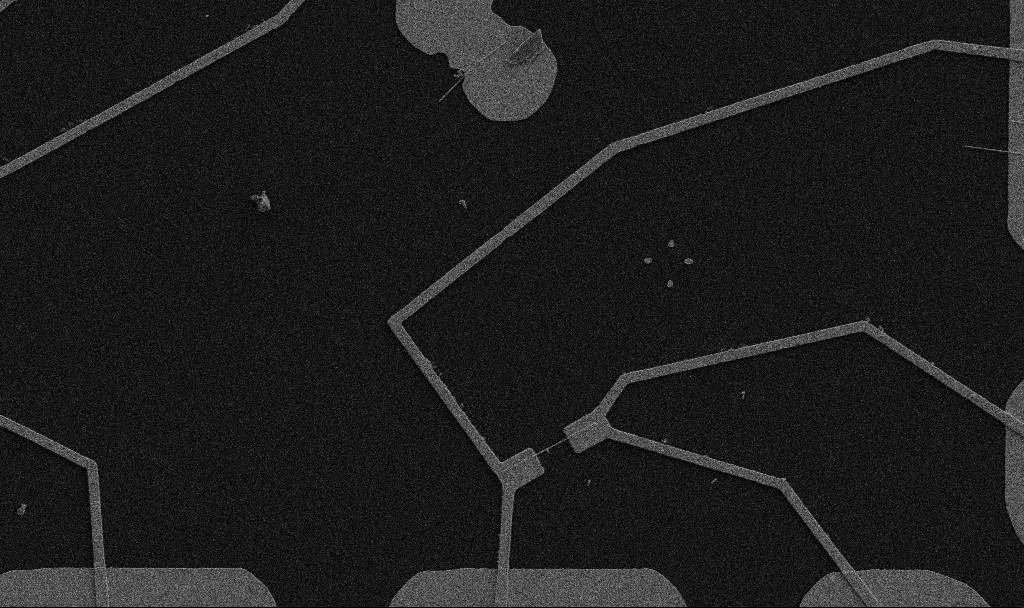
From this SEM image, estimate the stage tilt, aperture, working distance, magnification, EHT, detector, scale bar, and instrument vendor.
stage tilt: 0°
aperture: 30 µm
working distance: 10.7 mm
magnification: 5 K X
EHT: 5 kV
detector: SE2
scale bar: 10000 nm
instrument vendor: Zeiss